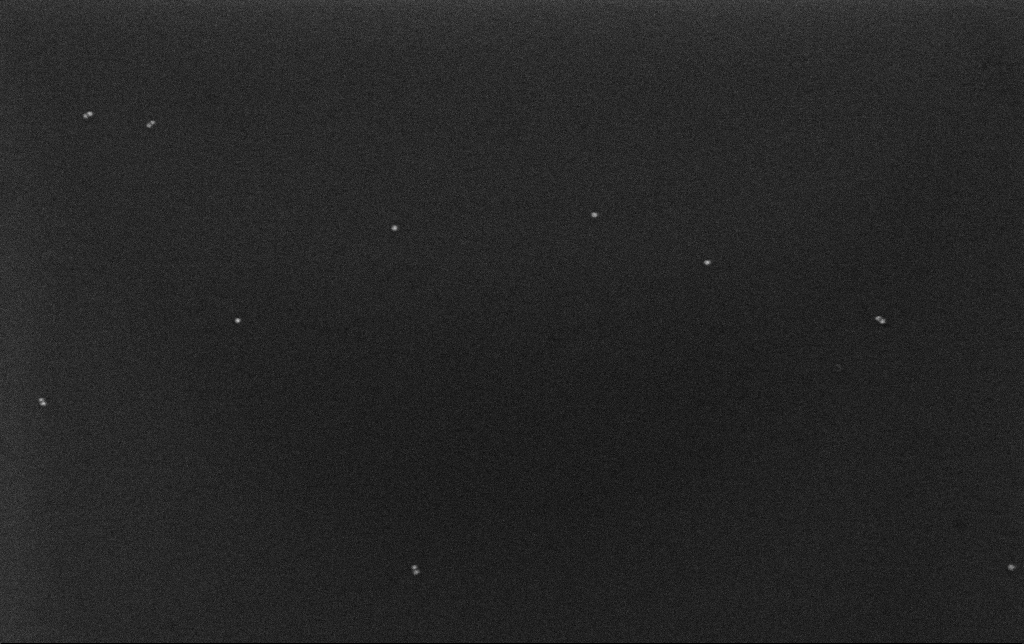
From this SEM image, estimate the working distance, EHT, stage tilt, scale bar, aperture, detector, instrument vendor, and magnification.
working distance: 3.2 mm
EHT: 10 kV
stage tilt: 0°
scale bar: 200 nm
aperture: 30 µm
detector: InLens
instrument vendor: Zeiss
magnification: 100 K X